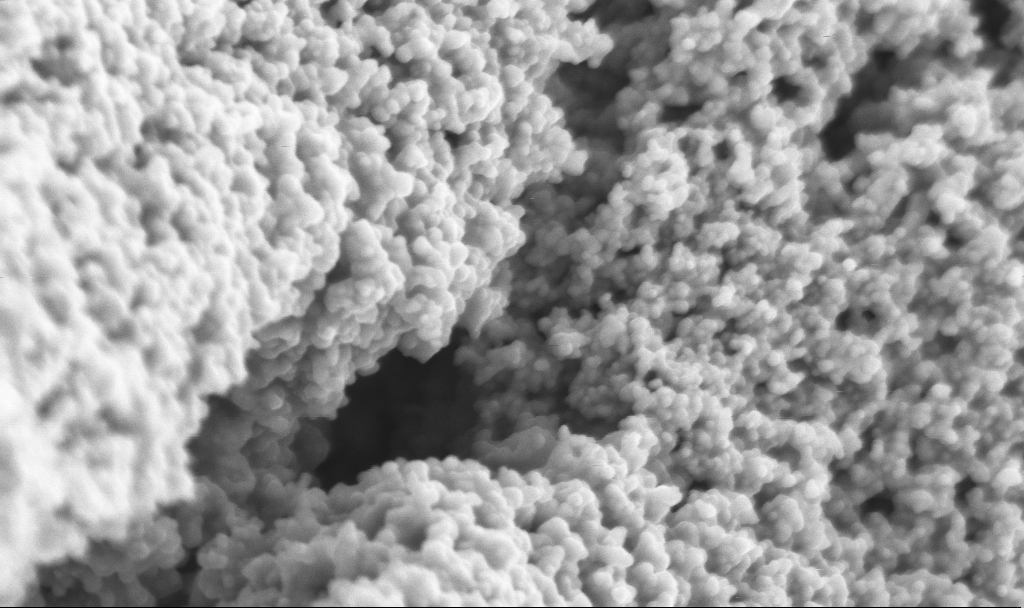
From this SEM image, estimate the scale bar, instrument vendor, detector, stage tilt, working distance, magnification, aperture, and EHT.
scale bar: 200 nm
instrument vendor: Zeiss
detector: InLens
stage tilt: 0°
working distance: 2.5 mm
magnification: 120.04 K X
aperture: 30 µm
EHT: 3 kV